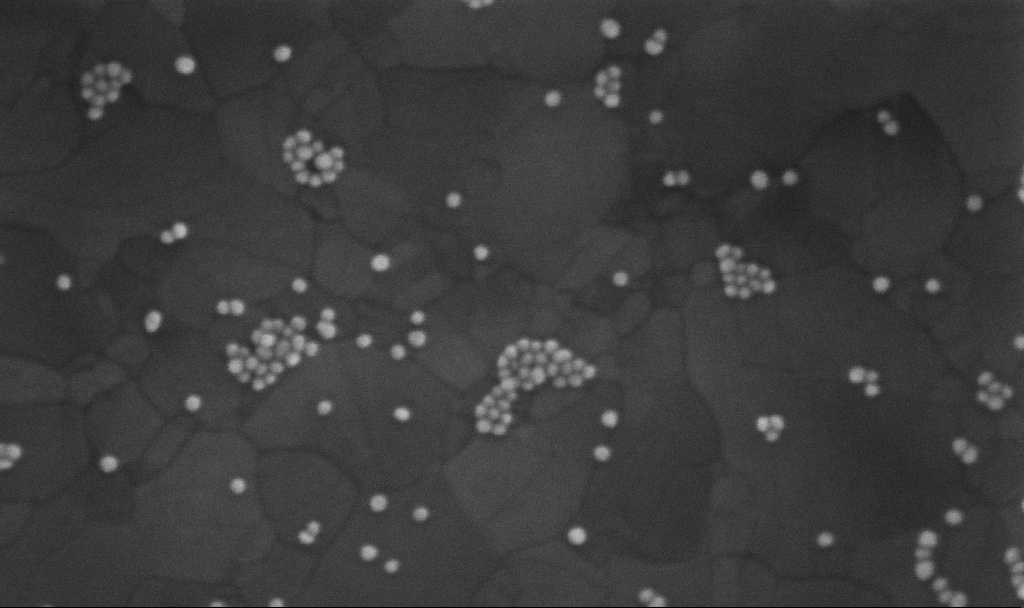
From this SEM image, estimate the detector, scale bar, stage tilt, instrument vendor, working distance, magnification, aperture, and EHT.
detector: InLens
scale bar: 200 nm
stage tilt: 0°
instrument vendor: Zeiss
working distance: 3.8 mm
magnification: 300 K X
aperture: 30 µm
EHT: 10 kV